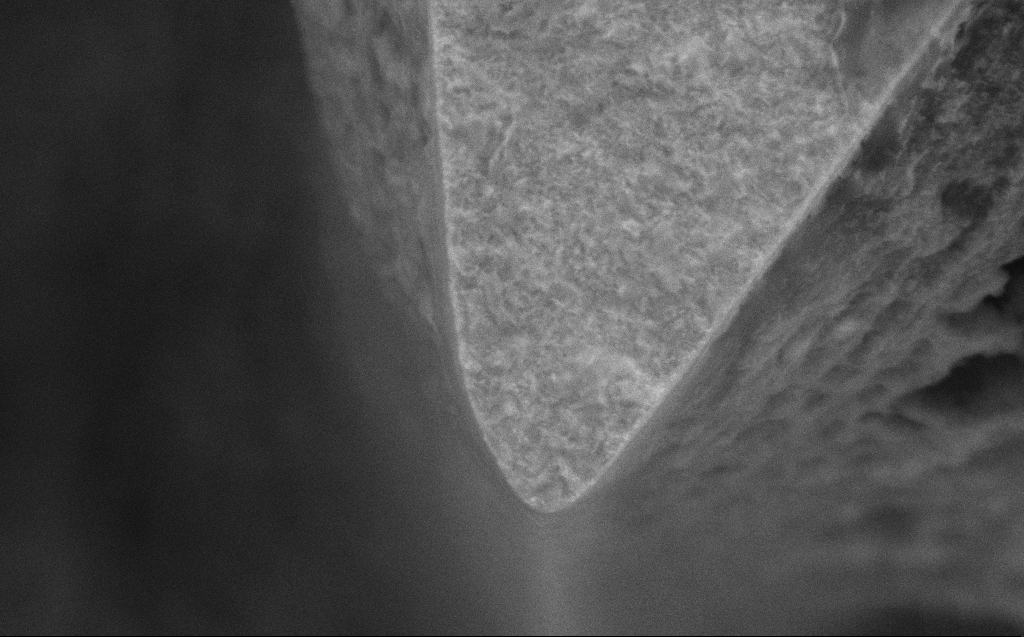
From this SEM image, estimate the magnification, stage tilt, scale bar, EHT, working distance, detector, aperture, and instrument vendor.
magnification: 40.42 K X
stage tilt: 0°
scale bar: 1000 nm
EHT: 5 kV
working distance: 7 mm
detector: SE2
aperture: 30 µm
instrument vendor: Zeiss